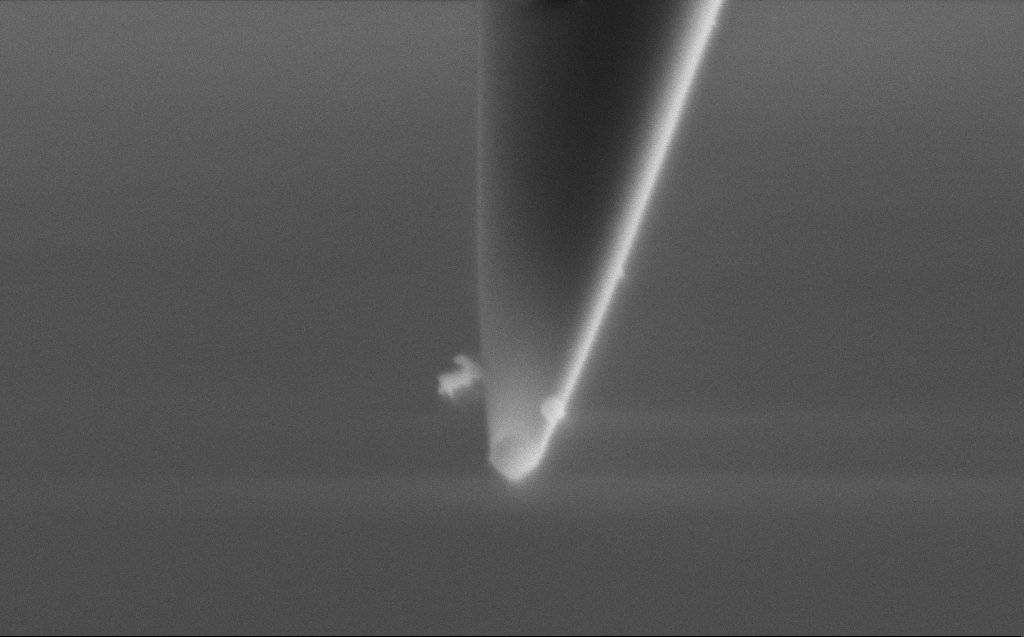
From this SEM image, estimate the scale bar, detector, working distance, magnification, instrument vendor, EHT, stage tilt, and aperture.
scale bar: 200 nm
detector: SE2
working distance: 5 mm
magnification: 146.02 K X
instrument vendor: Zeiss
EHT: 2 kV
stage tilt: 45.1°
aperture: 30 µm